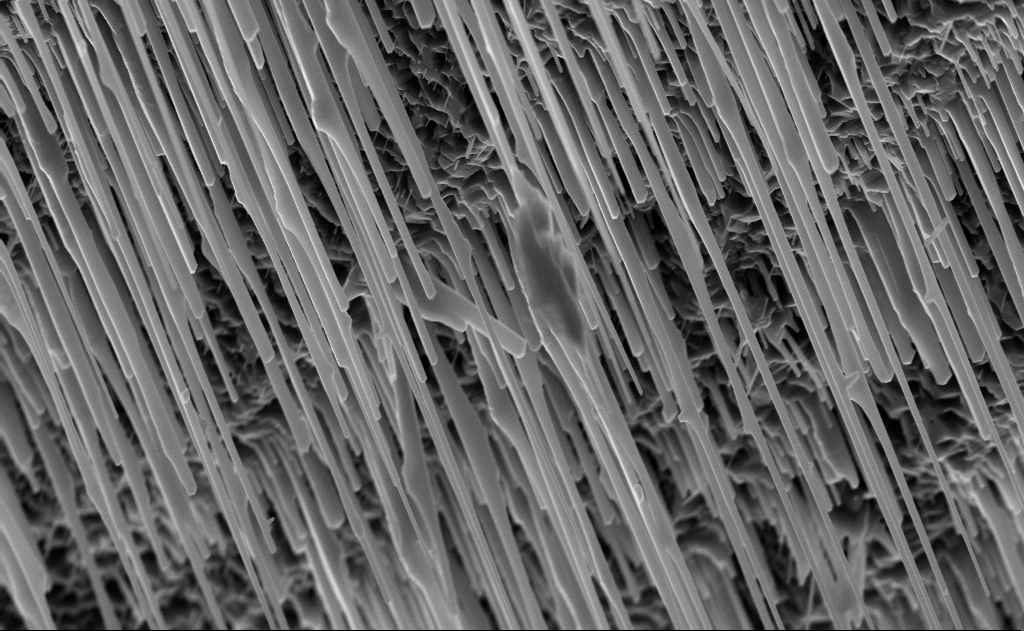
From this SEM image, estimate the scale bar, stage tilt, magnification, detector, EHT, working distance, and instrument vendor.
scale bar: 2000 nm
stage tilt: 0°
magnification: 20 K X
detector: InLens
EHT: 10 kV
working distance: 6 mm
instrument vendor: Zeiss